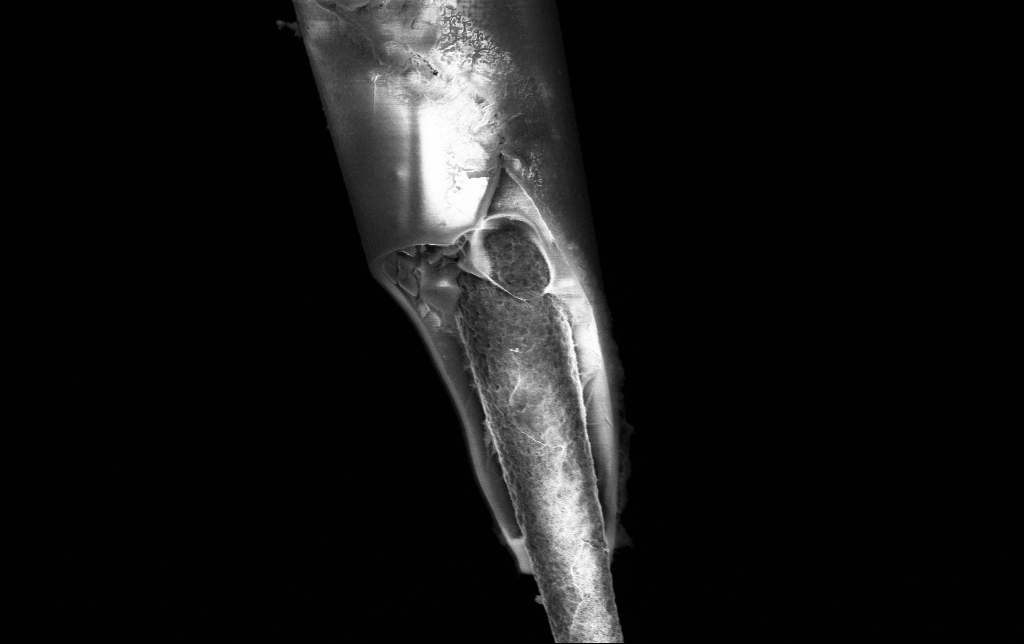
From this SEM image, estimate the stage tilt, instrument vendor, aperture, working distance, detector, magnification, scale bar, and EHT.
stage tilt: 0°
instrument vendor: Zeiss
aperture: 30 µm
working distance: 6.5 mm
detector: InLens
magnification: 20 K X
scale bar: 1000 nm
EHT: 3 kV